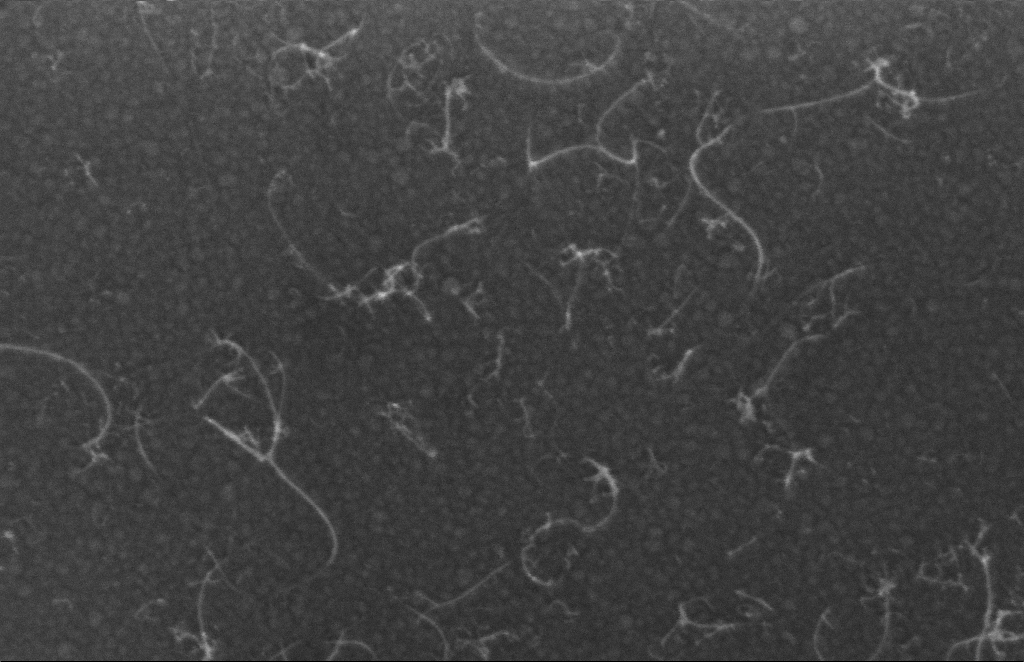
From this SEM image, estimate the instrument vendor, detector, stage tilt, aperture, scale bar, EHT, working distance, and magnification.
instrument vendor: Zeiss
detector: InLens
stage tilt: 0°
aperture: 20 µm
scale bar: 100 nm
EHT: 5 kV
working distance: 8 mm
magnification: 270.4 K X